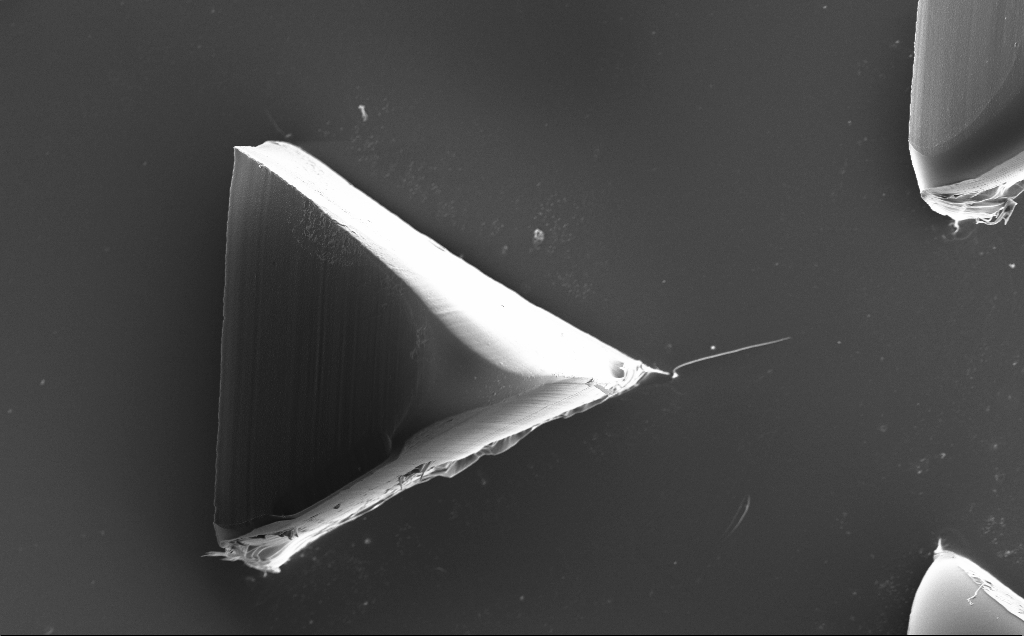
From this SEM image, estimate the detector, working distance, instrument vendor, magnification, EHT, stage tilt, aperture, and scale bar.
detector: InLens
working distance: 10 mm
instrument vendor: Zeiss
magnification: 0.632 K X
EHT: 10 kV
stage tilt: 0°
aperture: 30 µm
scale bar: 100000 nm